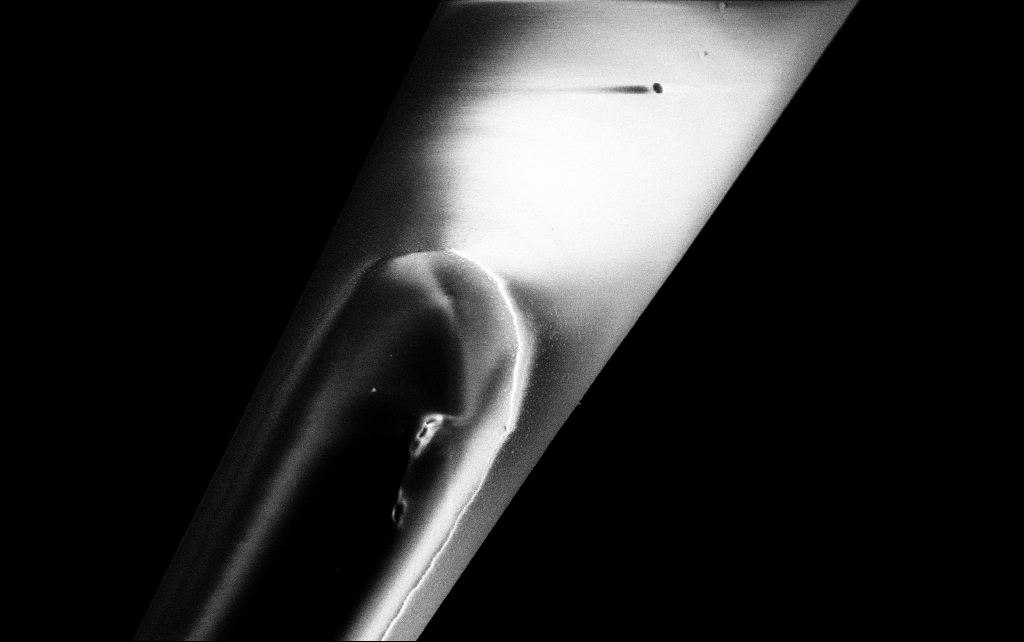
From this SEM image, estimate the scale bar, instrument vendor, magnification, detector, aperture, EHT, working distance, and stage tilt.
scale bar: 2000 nm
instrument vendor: Zeiss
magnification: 10 K X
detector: SE2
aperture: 30 µm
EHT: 3 kV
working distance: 7.6 mm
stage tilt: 45°